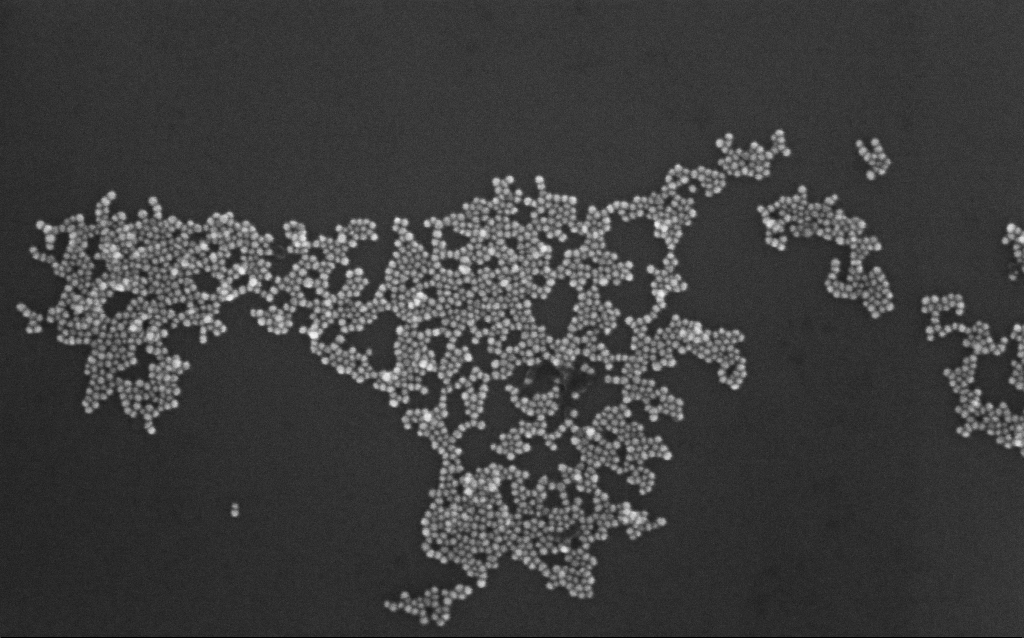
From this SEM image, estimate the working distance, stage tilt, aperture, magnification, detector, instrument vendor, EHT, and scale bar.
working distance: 7 mm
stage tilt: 0°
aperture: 30 µm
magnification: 164.72 K X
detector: InLens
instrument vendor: Zeiss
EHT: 10 kV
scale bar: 200 nm